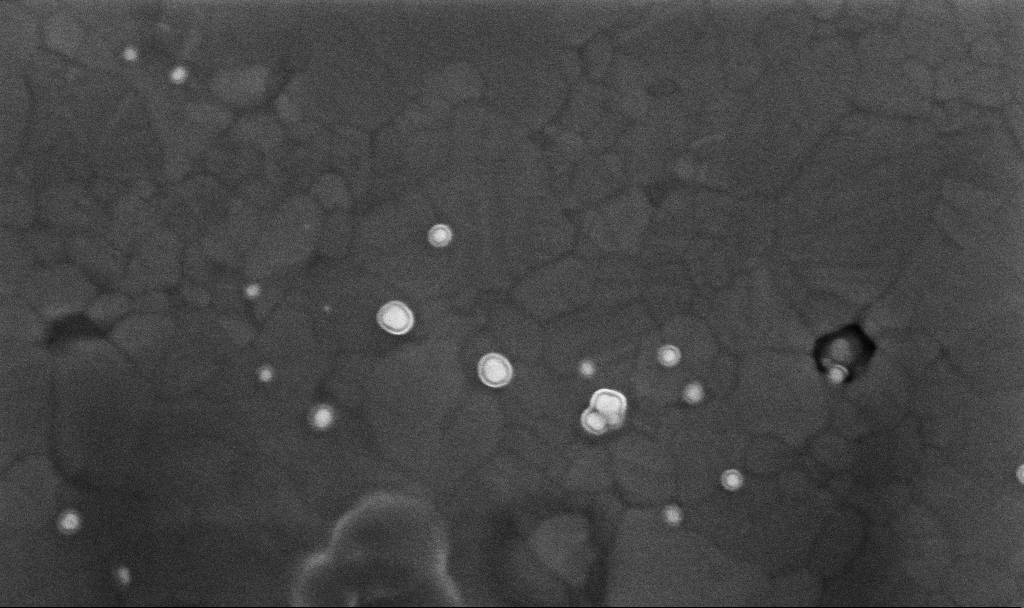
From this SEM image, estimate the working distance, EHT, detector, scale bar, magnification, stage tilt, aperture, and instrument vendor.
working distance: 3.4 mm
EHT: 10 kV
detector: InLens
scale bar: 200 nm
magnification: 169.89 K X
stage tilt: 0°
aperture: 30 µm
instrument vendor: Zeiss